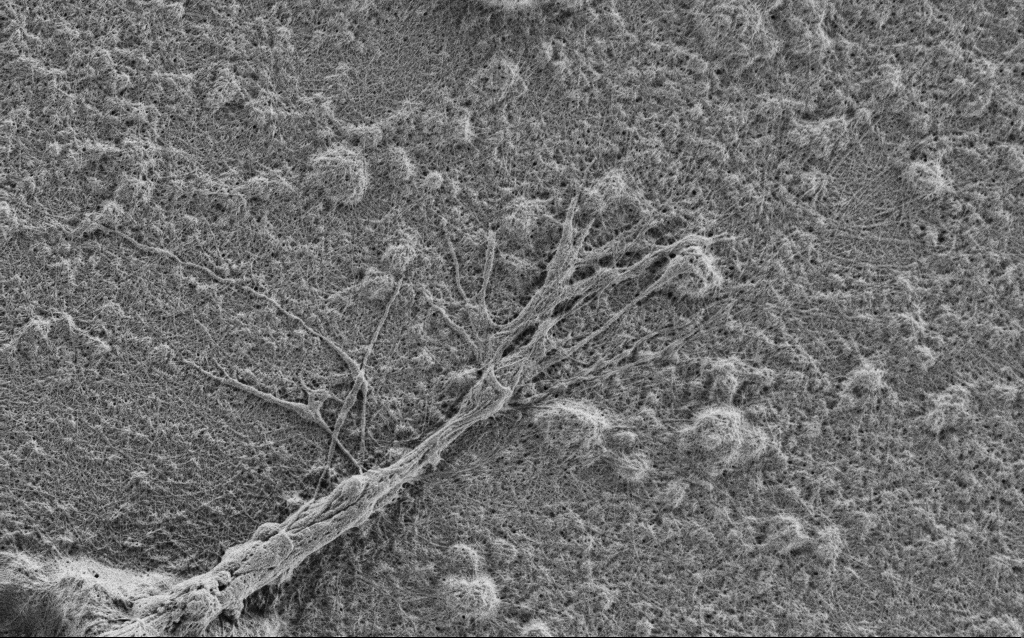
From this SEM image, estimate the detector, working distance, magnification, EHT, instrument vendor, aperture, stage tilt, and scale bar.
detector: SE2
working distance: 4 mm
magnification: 10 K X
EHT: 0.9 kV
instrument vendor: Zeiss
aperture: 30 µm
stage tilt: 0°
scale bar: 2000 nm